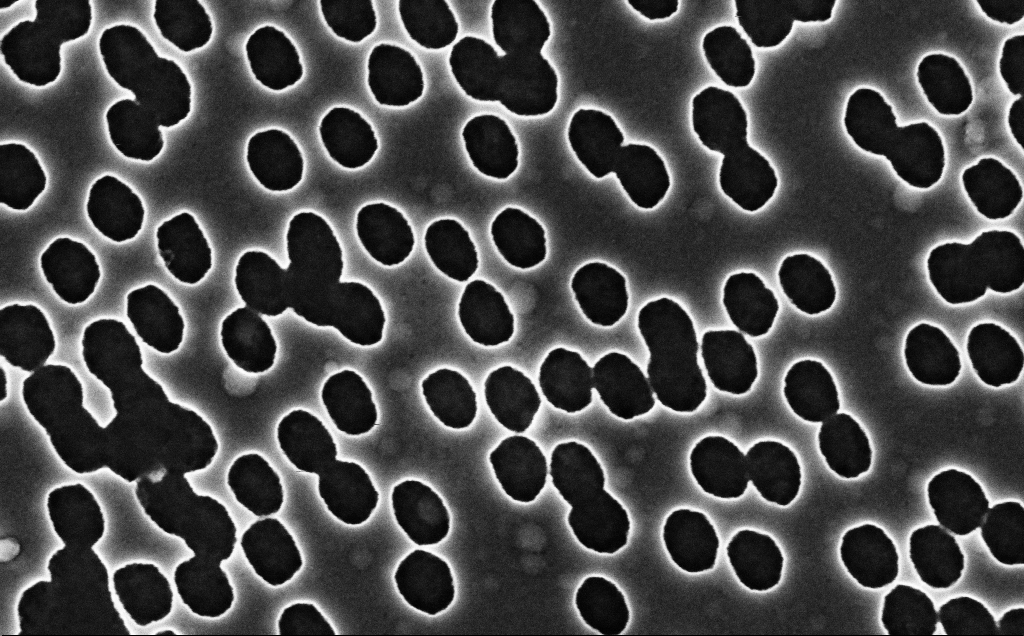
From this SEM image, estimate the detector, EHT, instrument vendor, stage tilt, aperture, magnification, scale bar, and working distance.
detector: InLens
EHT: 3 kV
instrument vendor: Zeiss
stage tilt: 0°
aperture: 30 µm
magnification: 90 K X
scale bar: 200 nm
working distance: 2.4 mm